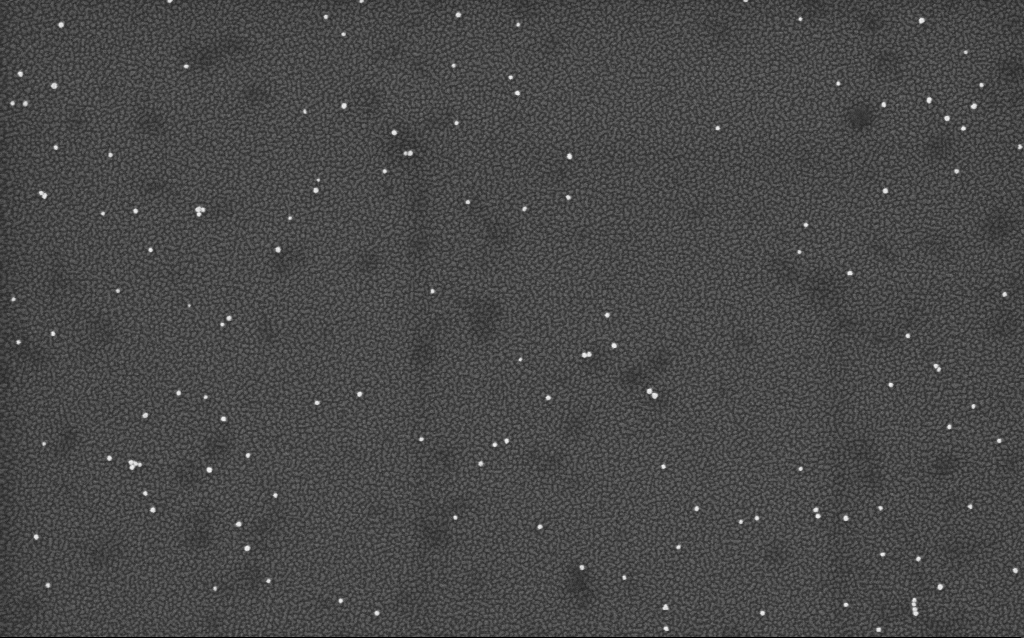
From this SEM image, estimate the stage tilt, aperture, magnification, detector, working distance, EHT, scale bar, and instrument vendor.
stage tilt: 0°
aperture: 30 µm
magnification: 100 K X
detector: InLens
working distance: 1.7 mm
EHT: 2 kV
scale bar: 200 nm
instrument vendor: Zeiss